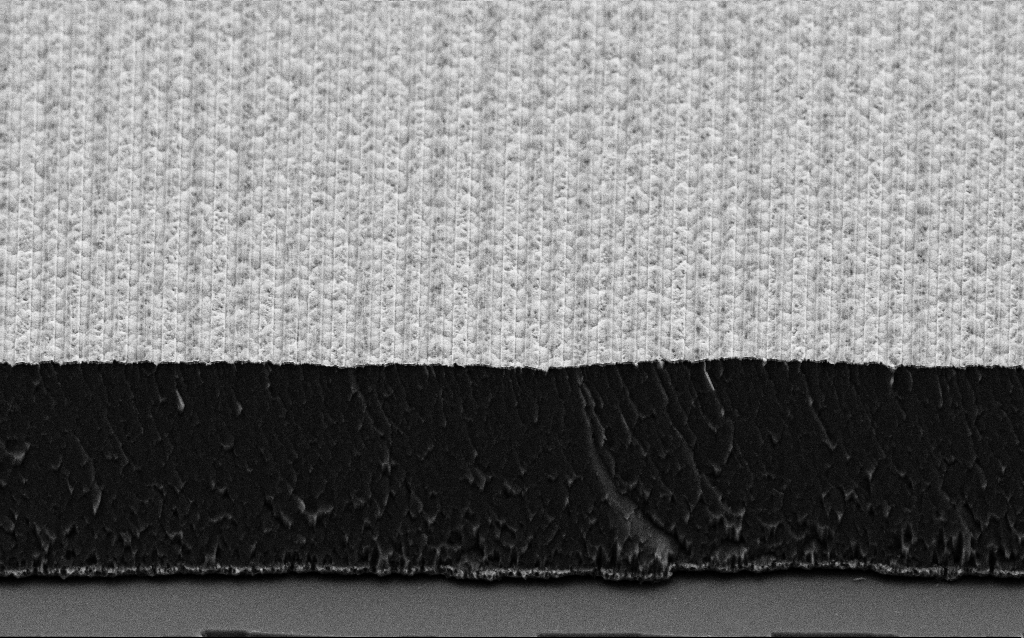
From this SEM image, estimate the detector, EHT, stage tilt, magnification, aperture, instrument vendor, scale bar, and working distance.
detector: SE2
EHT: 3 kV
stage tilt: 45°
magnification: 17.98 K X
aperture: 30 µm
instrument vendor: Zeiss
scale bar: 1000 nm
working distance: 6 mm